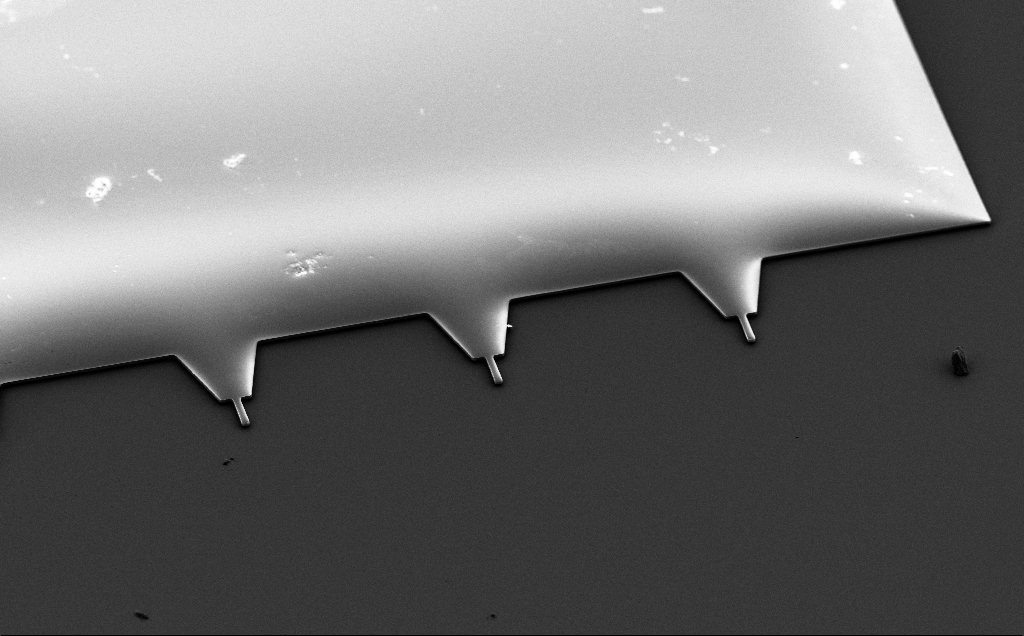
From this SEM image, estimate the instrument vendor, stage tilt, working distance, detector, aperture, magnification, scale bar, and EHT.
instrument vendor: Zeiss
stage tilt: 50°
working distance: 10 mm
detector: SE2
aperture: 30 µm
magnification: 0.6 K X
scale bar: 100000 nm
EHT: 5 kV